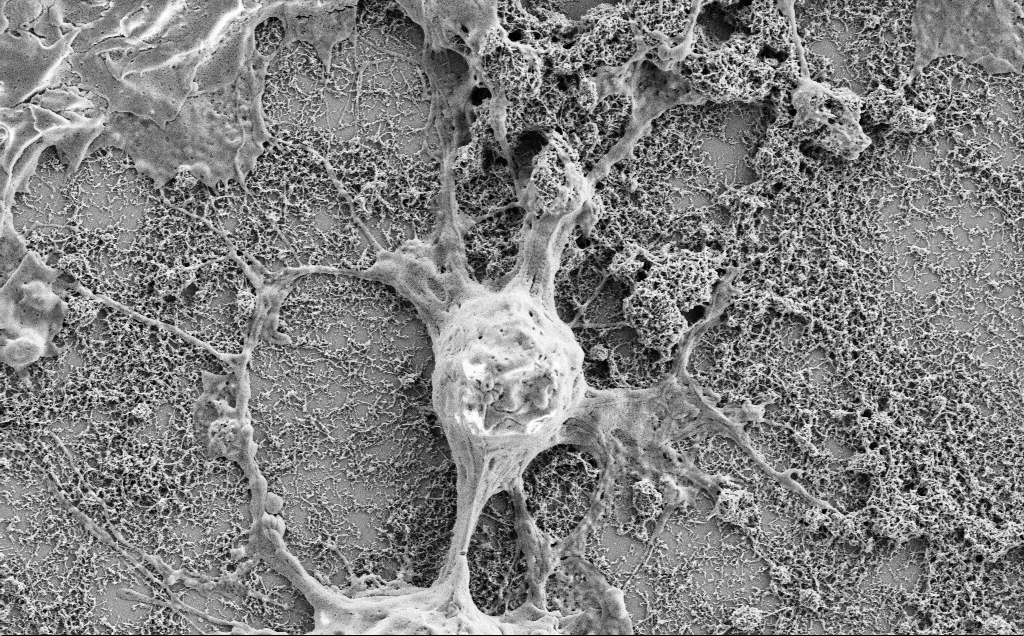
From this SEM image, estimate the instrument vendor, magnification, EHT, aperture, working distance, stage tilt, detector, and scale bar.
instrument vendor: Zeiss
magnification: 10 K X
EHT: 2 kV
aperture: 30 µm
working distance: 7.1 mm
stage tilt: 0°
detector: SE2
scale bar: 2000 nm